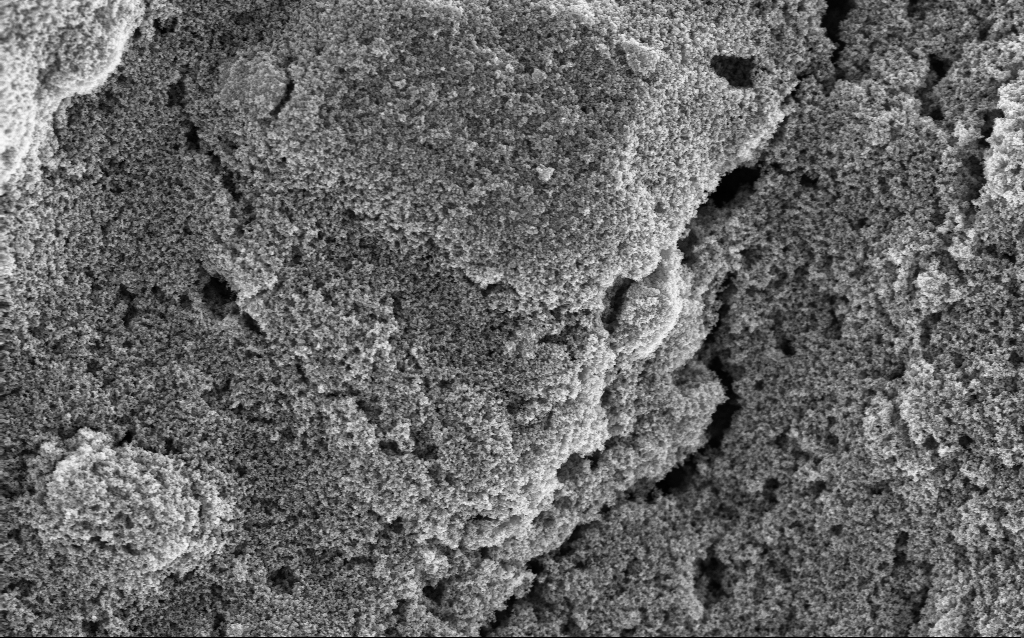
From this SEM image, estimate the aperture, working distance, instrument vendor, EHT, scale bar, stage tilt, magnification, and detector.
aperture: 30 µm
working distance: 3 mm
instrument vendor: Zeiss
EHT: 5 kV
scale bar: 1000 nm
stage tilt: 0°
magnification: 23.9 K X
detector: InLens